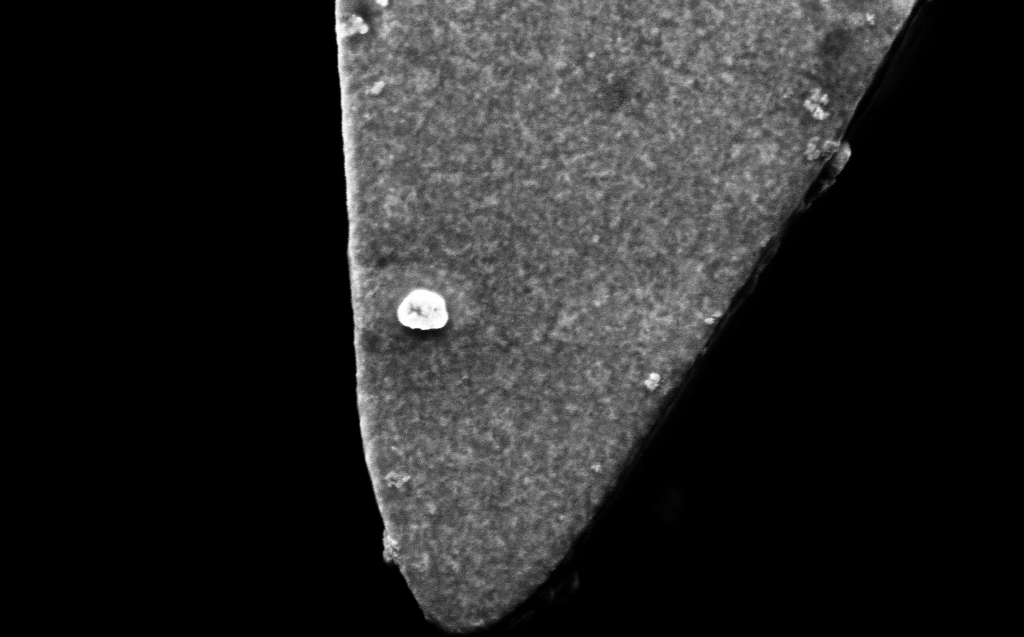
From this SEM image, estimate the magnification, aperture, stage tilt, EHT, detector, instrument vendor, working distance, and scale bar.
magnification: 54.98 K X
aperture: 30 µm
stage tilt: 0°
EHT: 3 kV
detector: InLens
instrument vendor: Zeiss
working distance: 4 mm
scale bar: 1000 nm